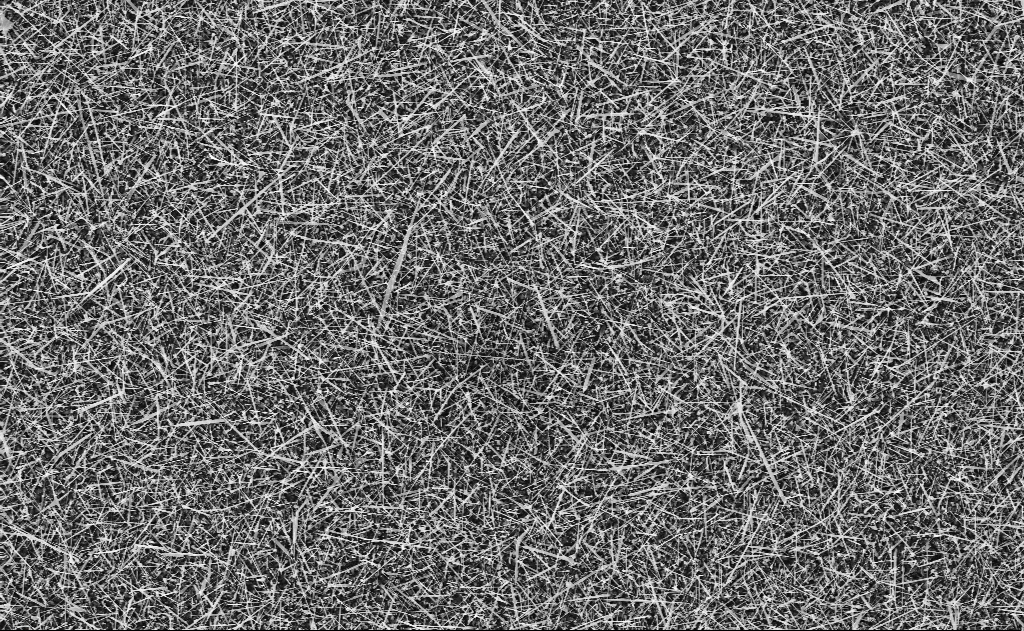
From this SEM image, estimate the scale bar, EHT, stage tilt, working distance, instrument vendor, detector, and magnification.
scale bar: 10000 nm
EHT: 10 kV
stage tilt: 0°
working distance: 11 mm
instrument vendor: Zeiss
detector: InLens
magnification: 5 K X